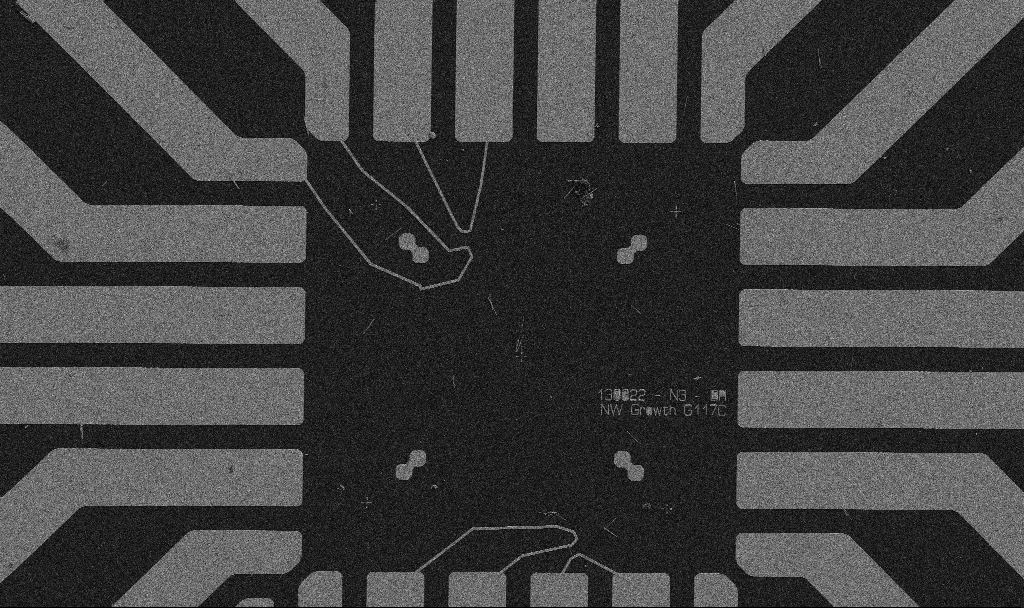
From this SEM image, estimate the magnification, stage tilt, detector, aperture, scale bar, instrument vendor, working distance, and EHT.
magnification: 1 K X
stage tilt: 0°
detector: SE2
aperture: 30 µm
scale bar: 20000 nm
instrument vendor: Zeiss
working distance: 10.7 mm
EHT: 5 kV